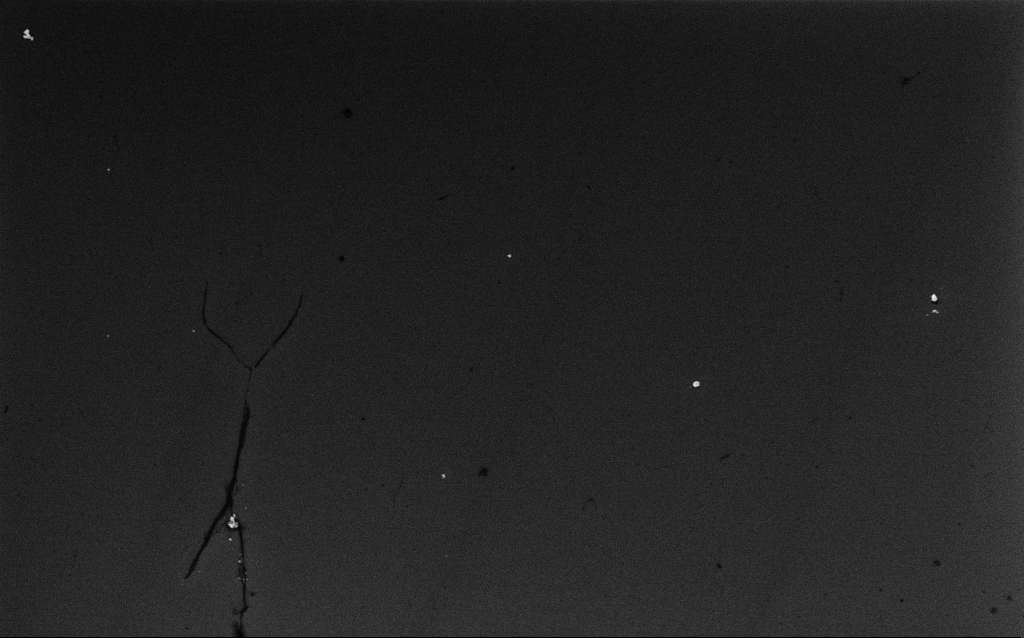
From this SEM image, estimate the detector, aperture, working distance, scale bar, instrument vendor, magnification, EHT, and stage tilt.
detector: InLens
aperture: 30 µm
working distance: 3.1 mm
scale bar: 10000 nm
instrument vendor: Zeiss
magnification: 3.97 K X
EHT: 10 kV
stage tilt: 0°